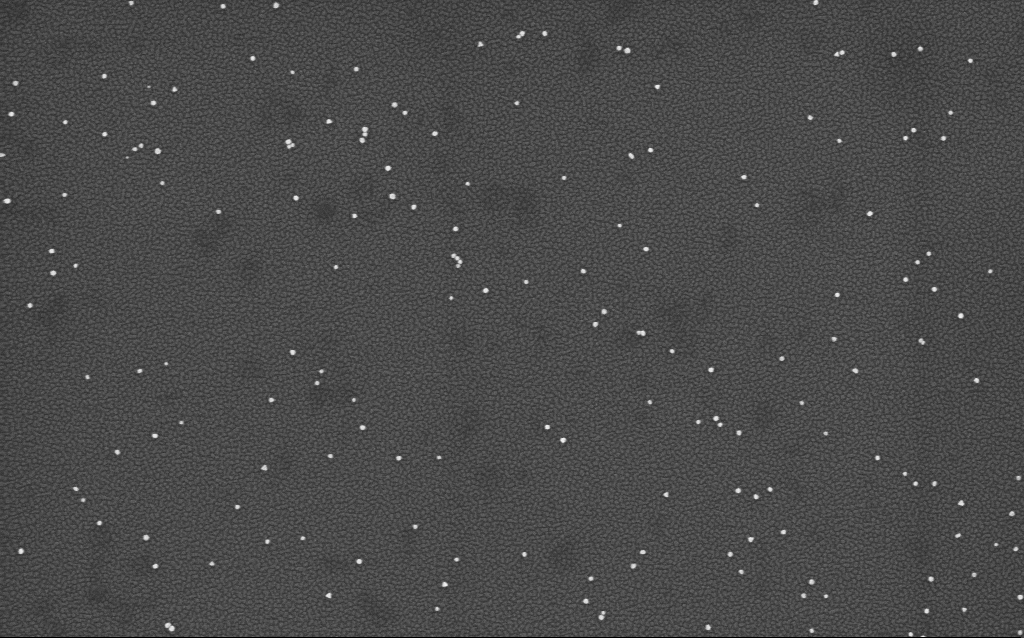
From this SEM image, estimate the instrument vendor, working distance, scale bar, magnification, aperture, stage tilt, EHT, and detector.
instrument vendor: Zeiss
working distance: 1.7 mm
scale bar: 200 nm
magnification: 100 K X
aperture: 30 µm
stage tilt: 0°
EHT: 4 kV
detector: InLens